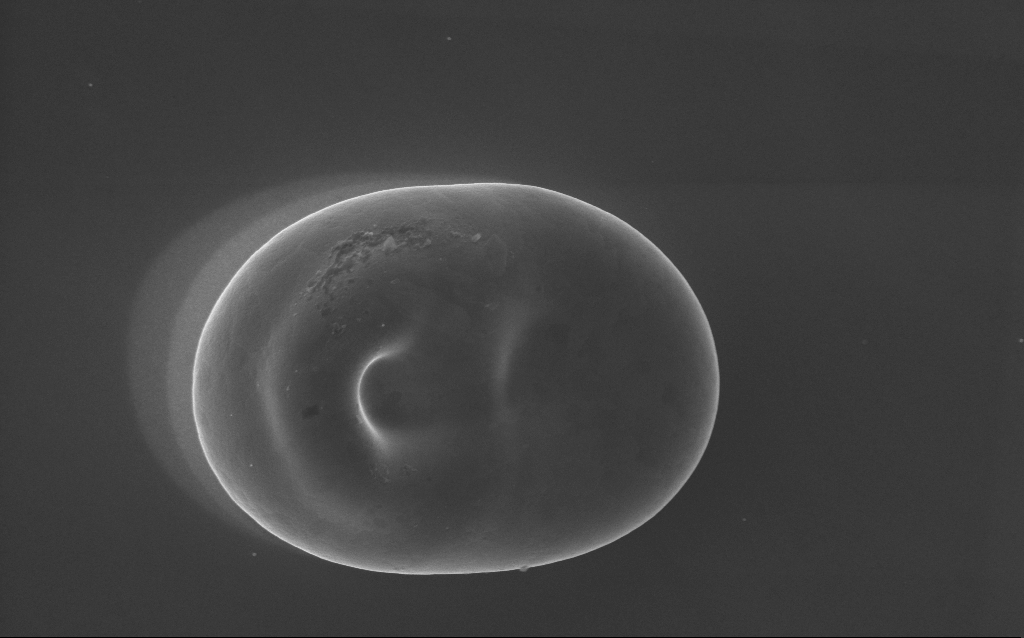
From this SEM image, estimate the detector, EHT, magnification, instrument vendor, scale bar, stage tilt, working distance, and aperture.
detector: InLens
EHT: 5 kV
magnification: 38 K X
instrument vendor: Zeiss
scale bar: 1000 nm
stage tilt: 0°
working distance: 3 mm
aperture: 30 µm